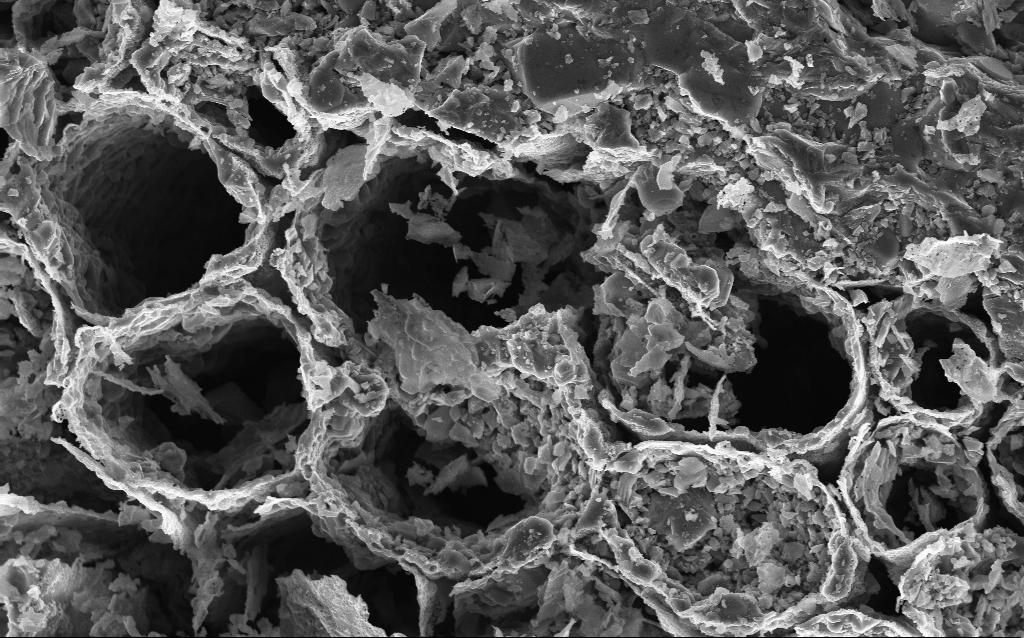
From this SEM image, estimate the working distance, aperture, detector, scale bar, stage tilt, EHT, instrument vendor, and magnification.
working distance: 3 mm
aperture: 30 µm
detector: InLens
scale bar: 10000 nm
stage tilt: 0°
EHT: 10 kV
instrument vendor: Zeiss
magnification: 5 K X